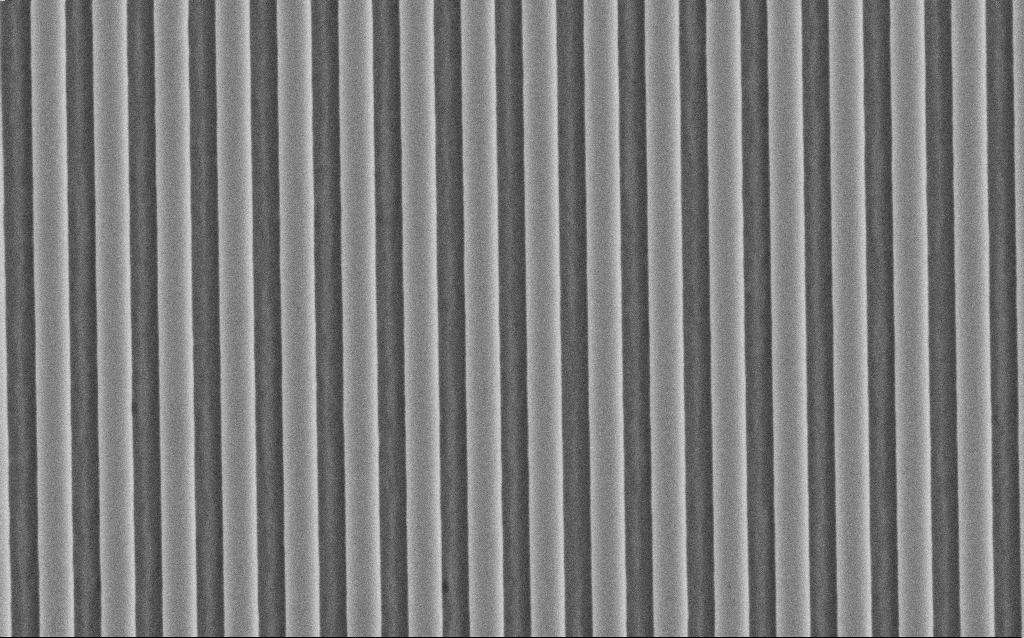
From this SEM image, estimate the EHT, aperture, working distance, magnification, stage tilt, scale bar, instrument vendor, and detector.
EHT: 3 kV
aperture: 30 µm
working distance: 5 mm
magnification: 45.52 K X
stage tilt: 0°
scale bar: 1000 nm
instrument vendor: Zeiss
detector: SE2